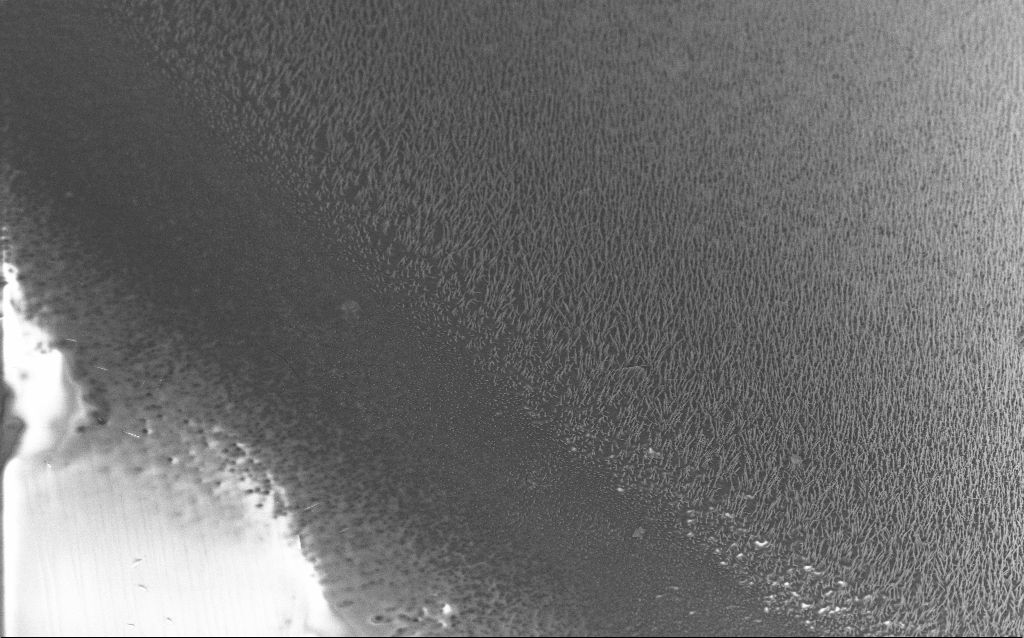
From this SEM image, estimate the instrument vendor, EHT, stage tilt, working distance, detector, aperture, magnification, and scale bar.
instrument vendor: Zeiss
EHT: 5 kV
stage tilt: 40°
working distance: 6.3 mm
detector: InLens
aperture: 30 µm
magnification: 1.5 K X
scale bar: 20000 nm